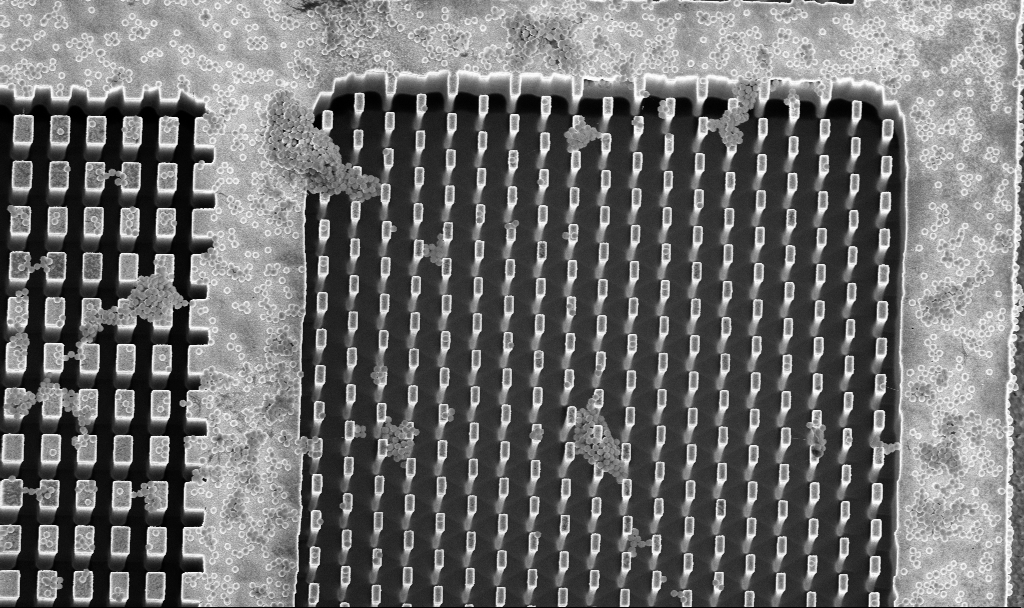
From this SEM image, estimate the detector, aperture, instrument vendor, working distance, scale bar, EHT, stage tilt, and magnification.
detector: InLens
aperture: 30 µm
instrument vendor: Zeiss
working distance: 3.3 mm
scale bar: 10000 nm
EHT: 5 kV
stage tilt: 8°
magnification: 4.19 K X